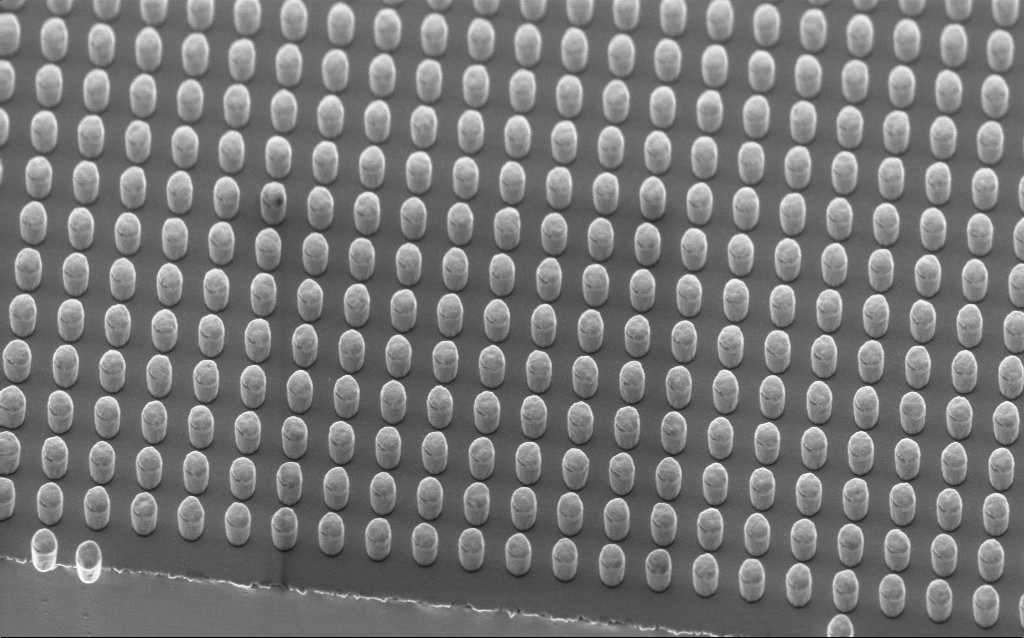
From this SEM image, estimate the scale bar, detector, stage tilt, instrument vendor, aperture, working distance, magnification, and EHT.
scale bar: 1000 nm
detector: InLens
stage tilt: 45°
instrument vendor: Zeiss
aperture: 30 µm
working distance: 3 mm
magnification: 34.76 K X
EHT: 2 kV